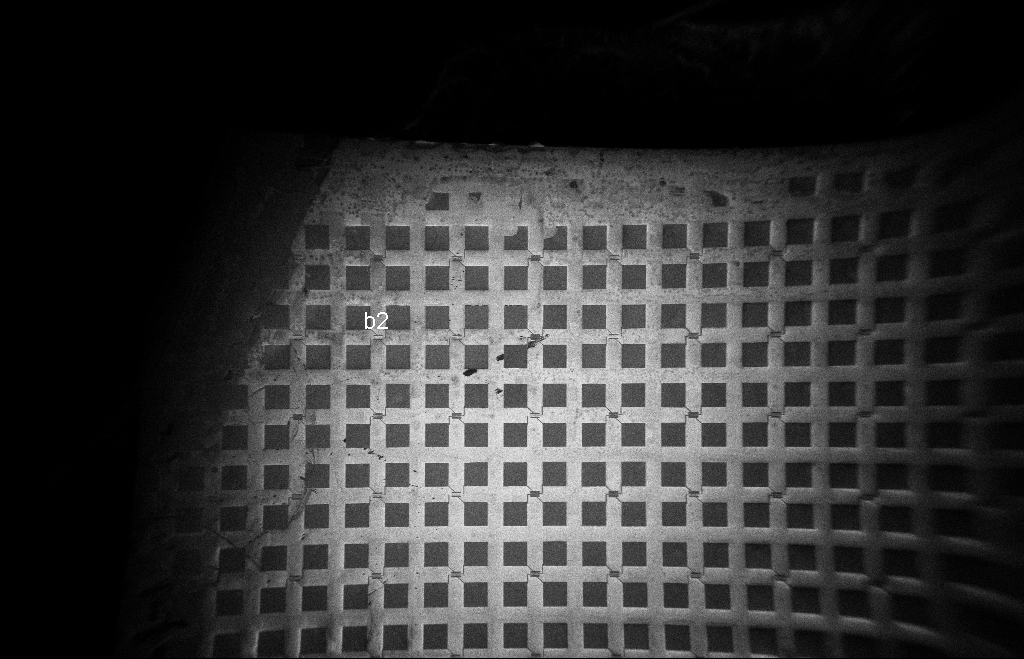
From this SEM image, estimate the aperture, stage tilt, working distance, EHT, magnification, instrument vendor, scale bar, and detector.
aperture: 20 µm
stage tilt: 0°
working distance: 12 mm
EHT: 5 kV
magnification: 0.058 K X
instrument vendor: Zeiss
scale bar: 200000 nm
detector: InLens